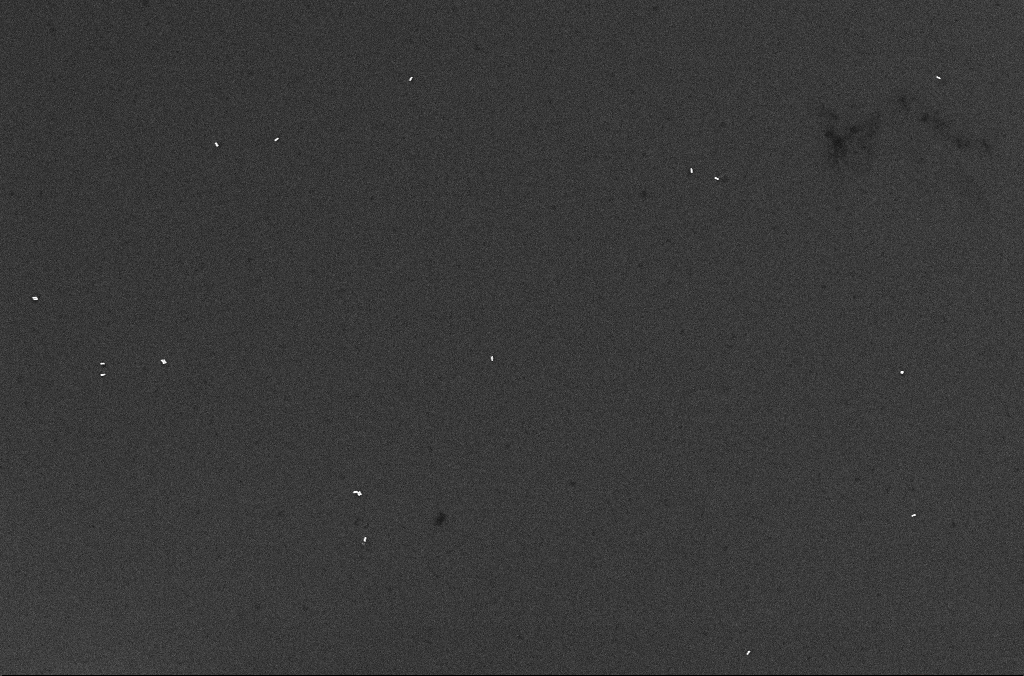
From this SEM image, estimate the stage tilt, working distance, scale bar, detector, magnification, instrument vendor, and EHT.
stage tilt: -0°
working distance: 2.5 mm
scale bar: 1000 nm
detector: InLens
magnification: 23.3 K X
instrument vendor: Zeiss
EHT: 10 kV